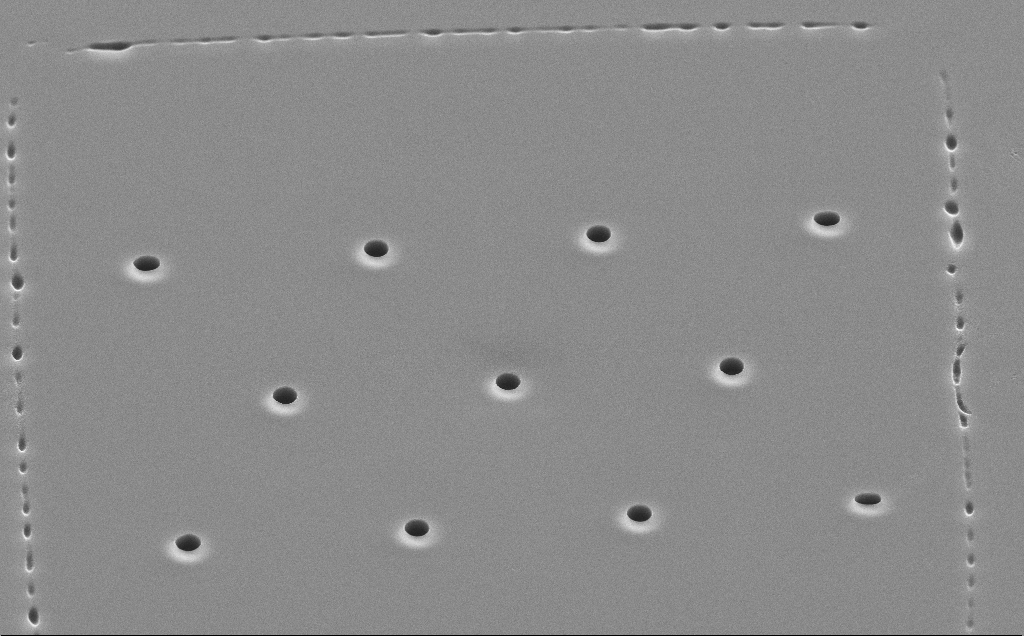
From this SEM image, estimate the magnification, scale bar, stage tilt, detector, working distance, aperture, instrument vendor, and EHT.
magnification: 4.08 K X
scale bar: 10000 nm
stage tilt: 45°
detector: SE2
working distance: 11 mm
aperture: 30 µm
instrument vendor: Zeiss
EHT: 10 kV